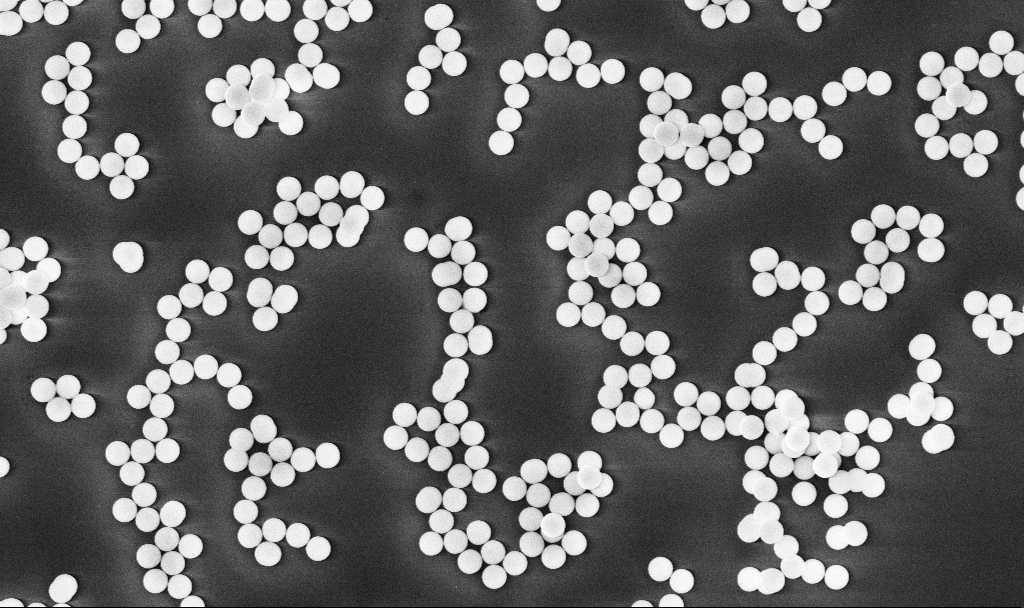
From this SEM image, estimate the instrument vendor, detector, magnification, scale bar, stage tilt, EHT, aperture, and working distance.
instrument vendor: Zeiss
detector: InLens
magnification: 7.07 K X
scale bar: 10000 nm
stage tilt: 0°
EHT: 10 kV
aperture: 30 µm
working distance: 5.4 mm